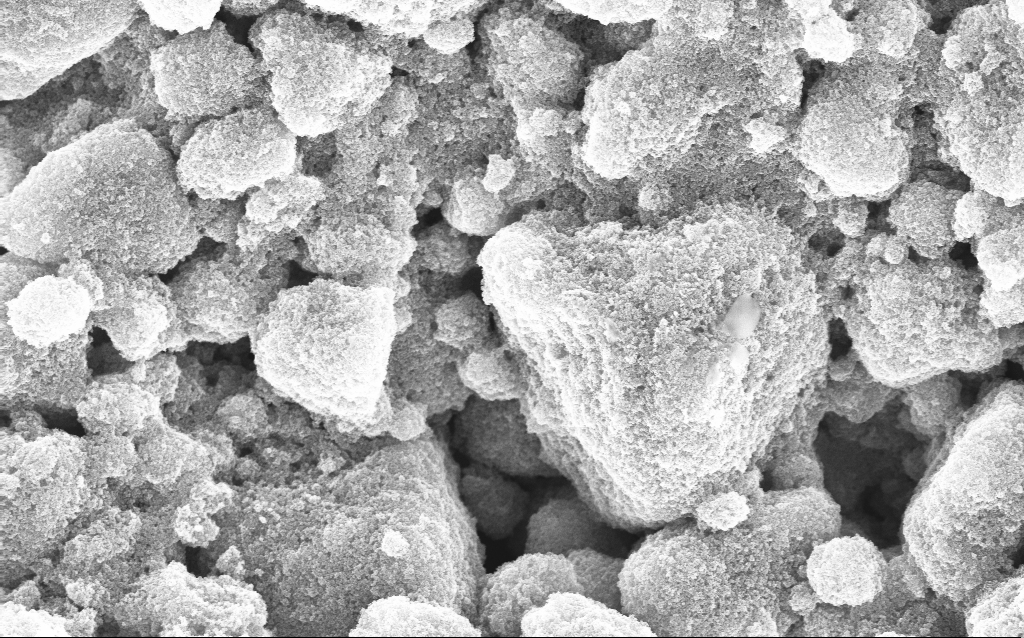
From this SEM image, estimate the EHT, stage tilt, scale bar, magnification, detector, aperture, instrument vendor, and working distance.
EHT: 10 kV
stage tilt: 0°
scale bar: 2000 nm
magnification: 19.77 K X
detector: InLens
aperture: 30 µm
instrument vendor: Zeiss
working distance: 3.8 mm